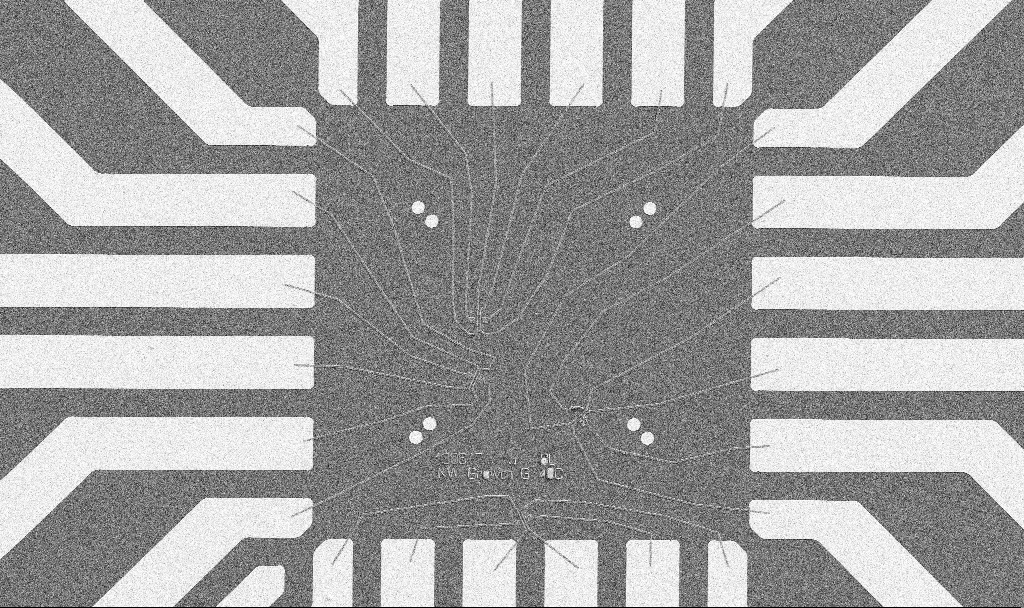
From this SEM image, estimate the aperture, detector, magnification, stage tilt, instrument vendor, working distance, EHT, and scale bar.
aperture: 30 µm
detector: SE2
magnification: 1 K X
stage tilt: -0°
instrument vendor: Zeiss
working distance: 10.7 mm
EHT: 5 kV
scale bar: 20000 nm